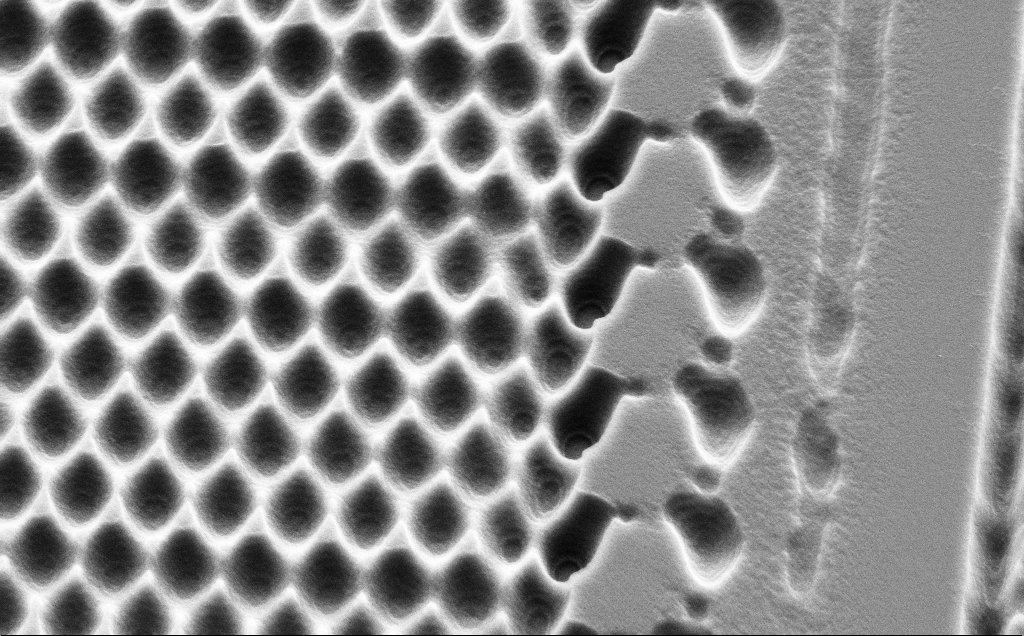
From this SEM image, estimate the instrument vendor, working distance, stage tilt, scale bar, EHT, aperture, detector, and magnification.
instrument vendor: Zeiss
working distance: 10 mm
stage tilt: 45°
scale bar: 2000 nm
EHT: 5 kV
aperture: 30 µm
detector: SE2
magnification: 13.07 K X